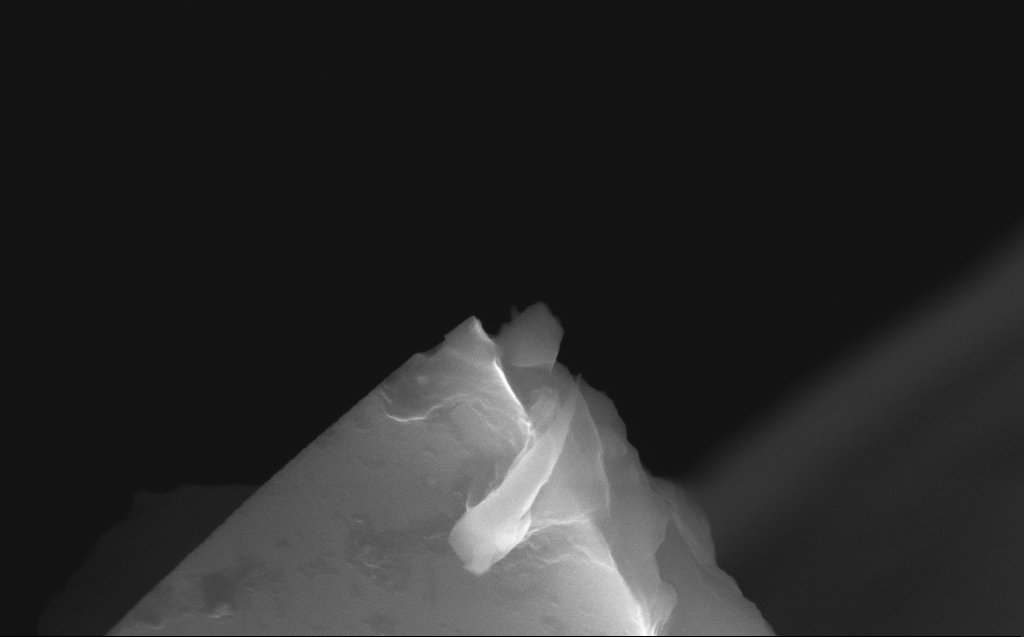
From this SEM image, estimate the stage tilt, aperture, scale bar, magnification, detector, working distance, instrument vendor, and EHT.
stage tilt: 35.7°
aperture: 30 µm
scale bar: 200 nm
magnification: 73.73 K X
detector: InLens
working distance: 6 mm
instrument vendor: Zeiss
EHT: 10 kV